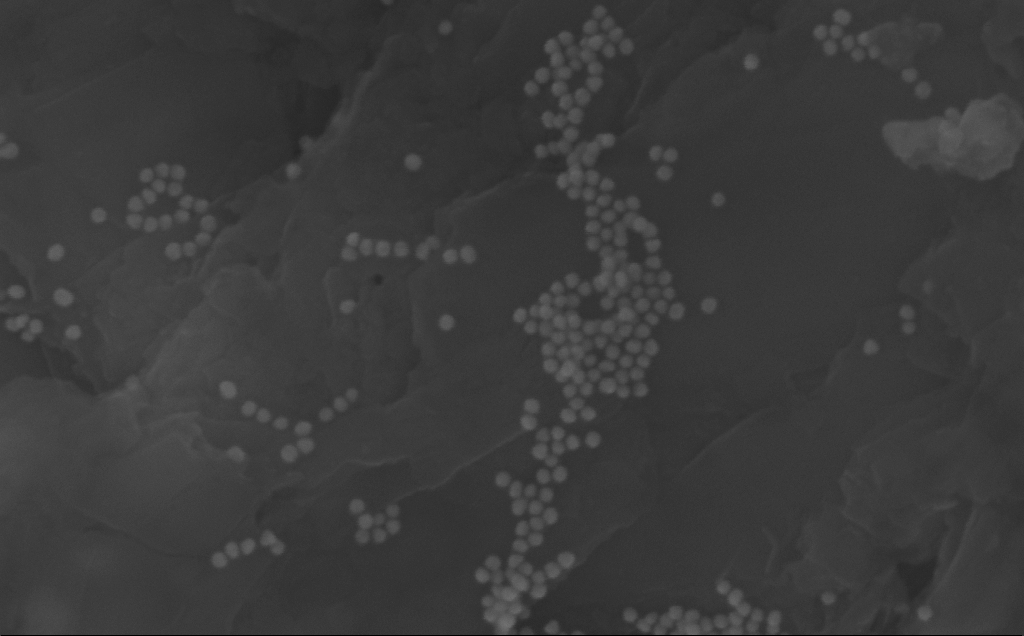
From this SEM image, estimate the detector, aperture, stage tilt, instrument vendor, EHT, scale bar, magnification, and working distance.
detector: InLens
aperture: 30 µm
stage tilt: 0°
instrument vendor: Zeiss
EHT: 10 kV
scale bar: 200 nm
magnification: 344.06 K X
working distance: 3 mm